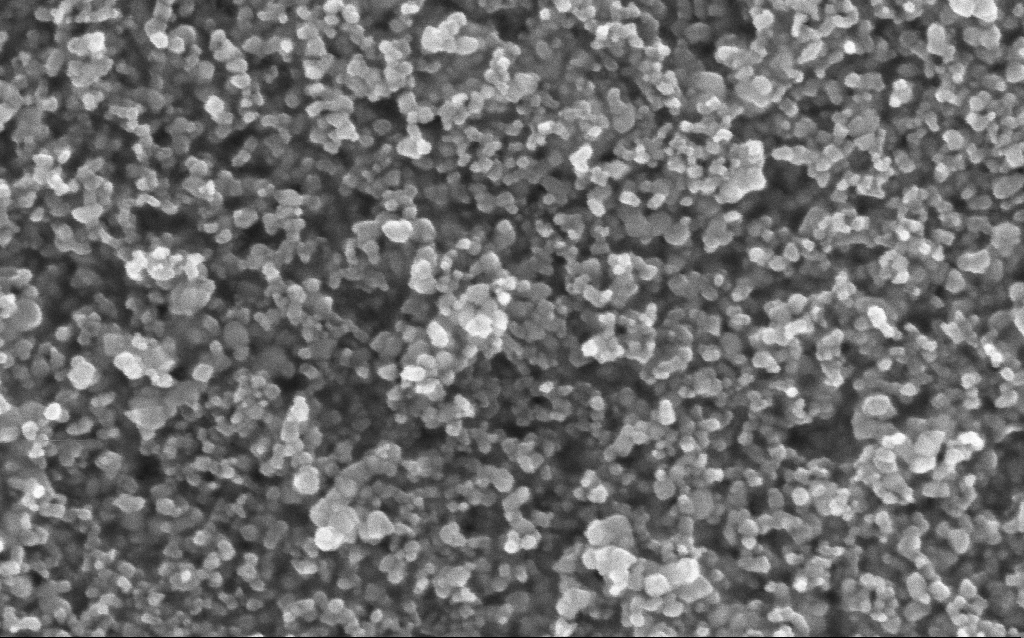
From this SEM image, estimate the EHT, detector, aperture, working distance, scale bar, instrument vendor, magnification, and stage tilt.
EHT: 5 kV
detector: InLens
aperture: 30 µm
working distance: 6.4 mm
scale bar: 100 nm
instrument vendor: Zeiss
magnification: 204.13 K X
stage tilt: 0°